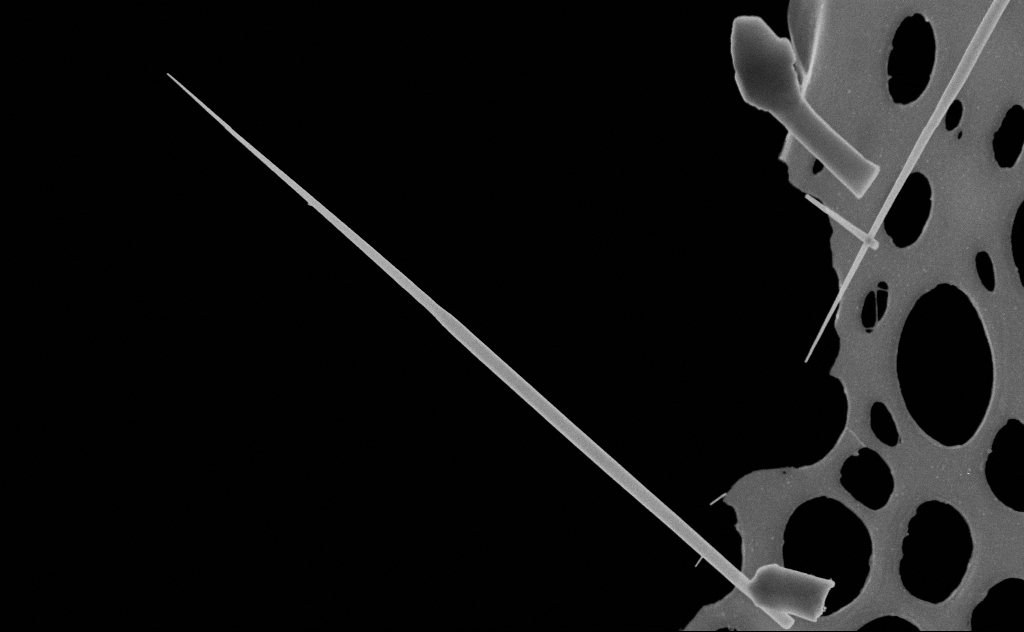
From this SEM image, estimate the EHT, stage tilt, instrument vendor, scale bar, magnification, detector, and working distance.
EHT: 20 kV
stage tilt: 0°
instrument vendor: Zeiss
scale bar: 1000 nm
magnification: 37.77 K X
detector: SE2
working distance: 10 mm